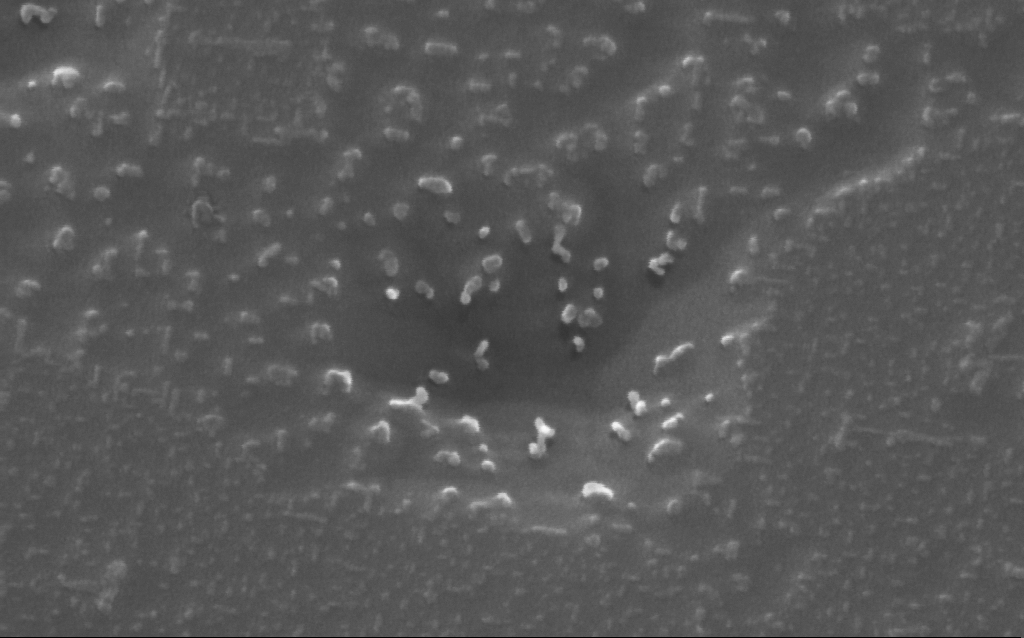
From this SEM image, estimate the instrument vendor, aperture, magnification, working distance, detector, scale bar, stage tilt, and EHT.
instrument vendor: Zeiss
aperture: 30 µm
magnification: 268.45 K X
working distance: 6 mm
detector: InLens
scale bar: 200 nm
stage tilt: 21.3°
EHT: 10 kV